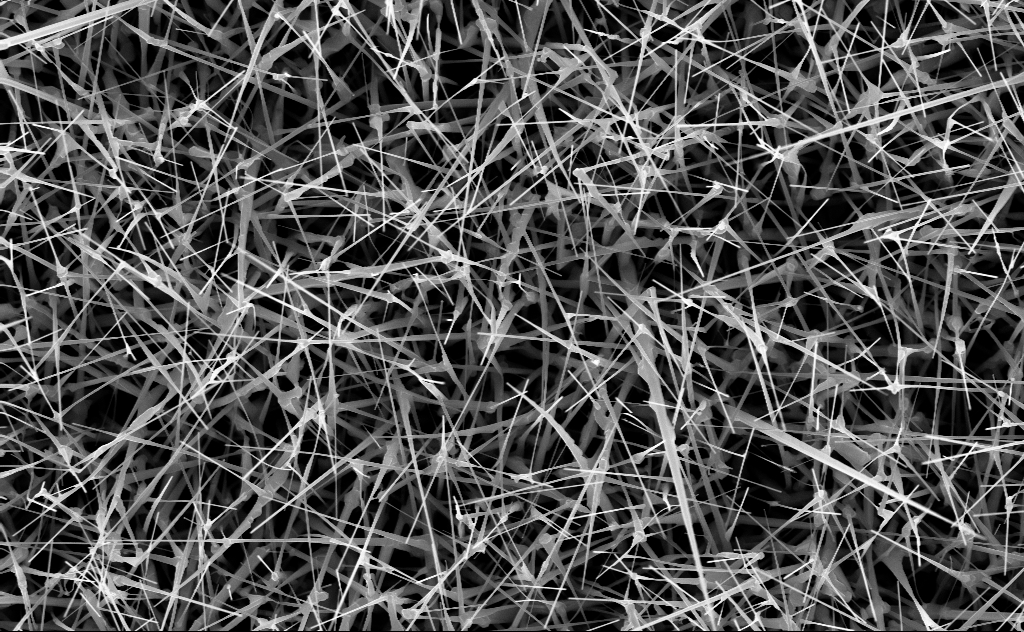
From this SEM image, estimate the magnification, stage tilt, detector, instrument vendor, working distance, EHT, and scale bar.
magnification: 20 K X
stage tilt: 0°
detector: InLens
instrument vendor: Zeiss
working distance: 6 mm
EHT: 10 kV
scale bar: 2000 nm